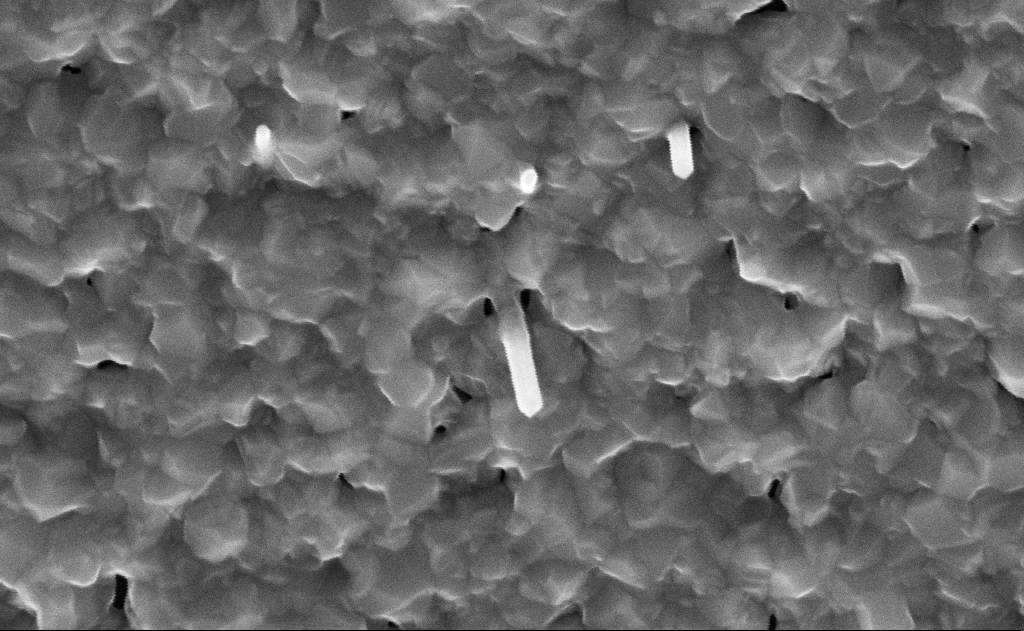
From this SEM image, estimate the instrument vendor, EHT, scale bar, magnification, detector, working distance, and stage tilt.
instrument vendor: Zeiss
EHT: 10 kV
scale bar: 200 nm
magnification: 100 K X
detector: InLens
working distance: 15 mm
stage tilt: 0°